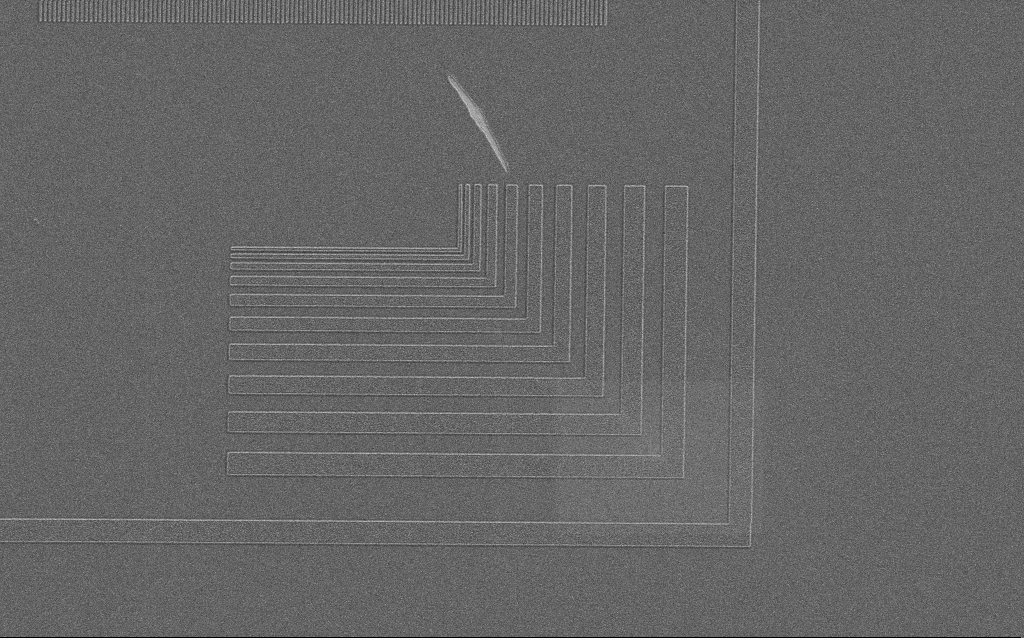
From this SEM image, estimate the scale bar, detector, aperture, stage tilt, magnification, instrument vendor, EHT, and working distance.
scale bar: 2000 nm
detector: SE2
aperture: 30 µm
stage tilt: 0°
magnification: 8.33 K X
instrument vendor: Zeiss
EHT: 1.5 kV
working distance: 6 mm